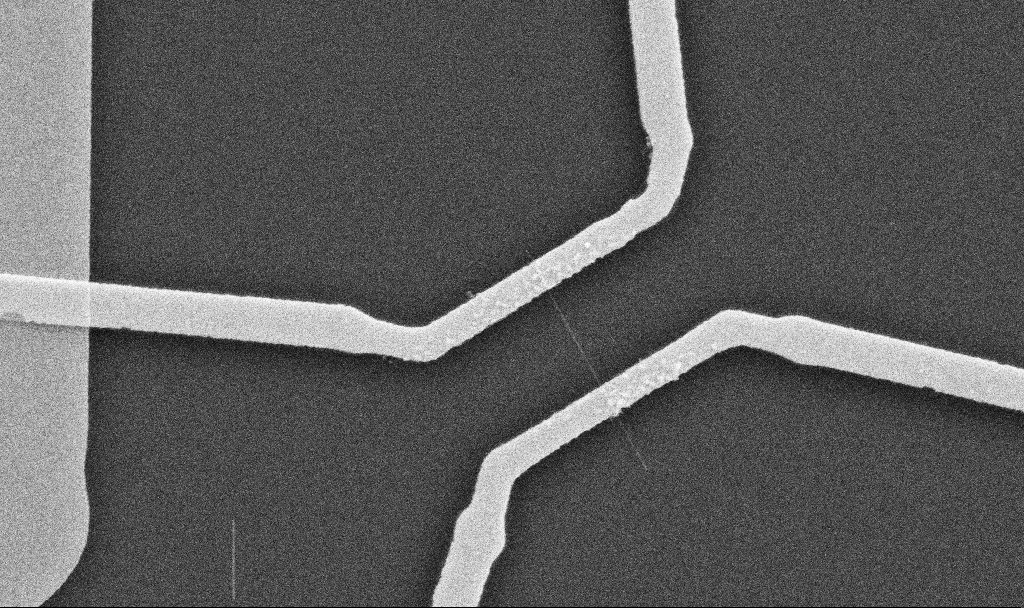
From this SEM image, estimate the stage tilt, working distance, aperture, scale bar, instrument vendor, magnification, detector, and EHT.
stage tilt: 0°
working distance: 10.7 mm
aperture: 30 µm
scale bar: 1000 nm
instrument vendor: Zeiss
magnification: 20 K X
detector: SE2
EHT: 10 kV